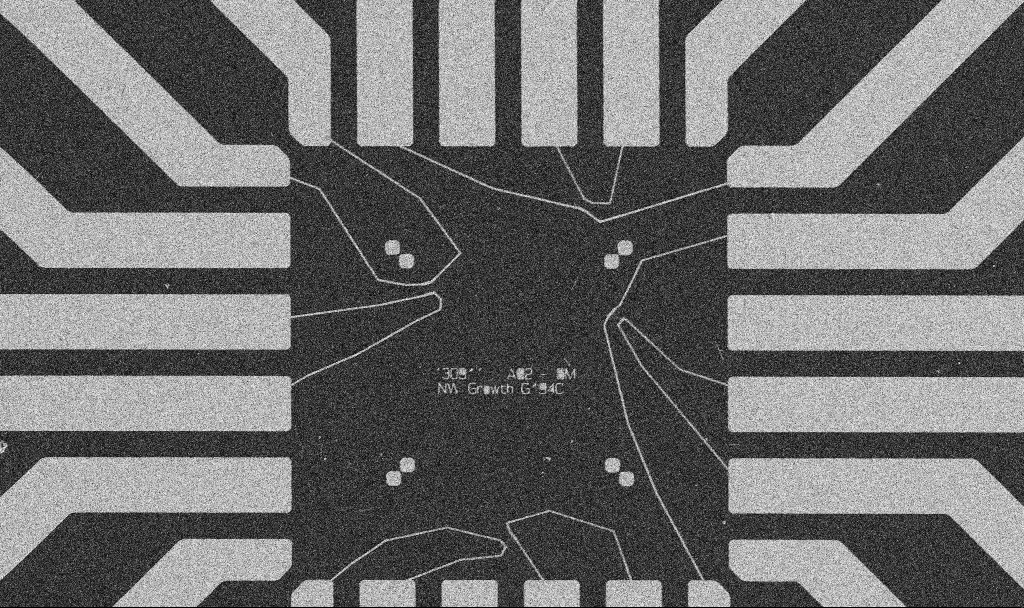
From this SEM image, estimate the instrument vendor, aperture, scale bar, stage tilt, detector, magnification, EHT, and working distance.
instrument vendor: Zeiss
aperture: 30 µm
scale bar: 20000 nm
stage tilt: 0°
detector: SE2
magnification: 1 K X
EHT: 5 kV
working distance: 10.7 mm